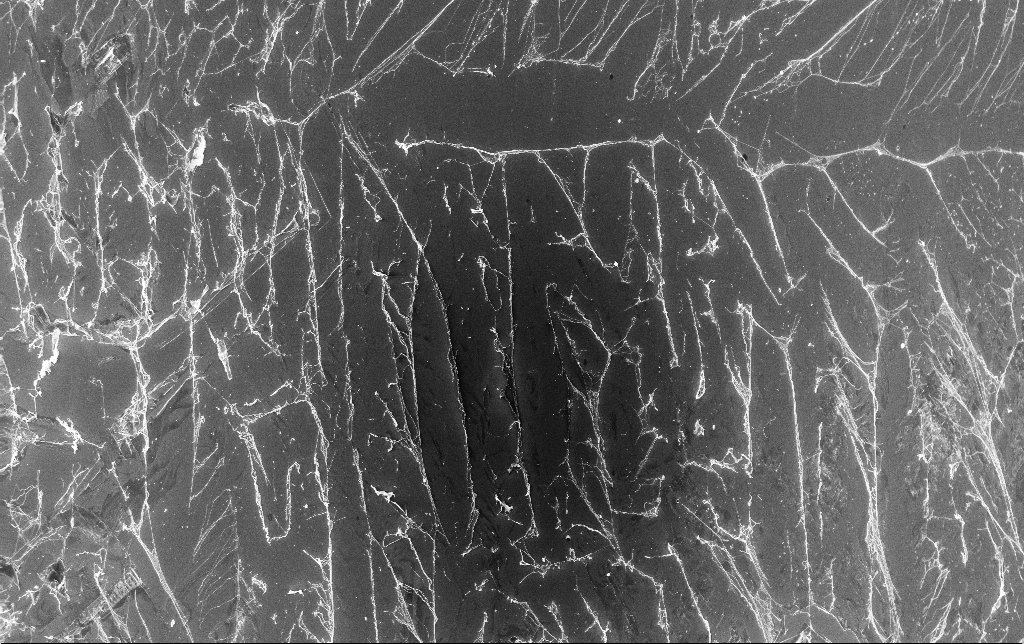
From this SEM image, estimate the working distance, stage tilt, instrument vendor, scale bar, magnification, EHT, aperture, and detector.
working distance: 3.4 mm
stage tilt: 0°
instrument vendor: Zeiss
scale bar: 100000 nm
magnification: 0.266 K X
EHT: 10 kV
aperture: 30 µm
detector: InLens